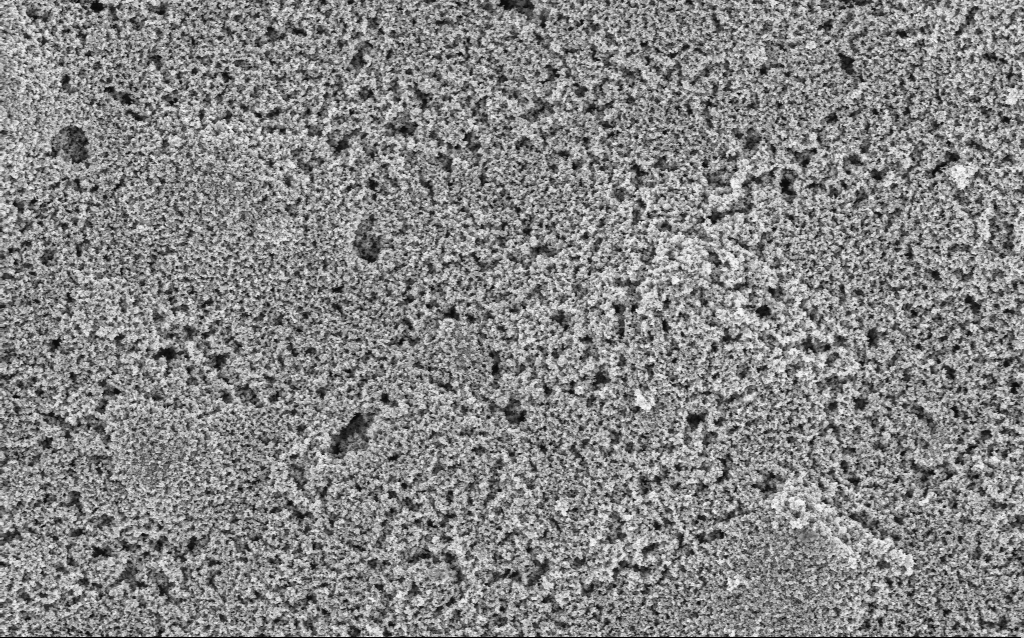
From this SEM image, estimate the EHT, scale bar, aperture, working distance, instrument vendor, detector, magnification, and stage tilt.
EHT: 3 kV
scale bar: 1000 nm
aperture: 30 µm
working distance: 7.6 mm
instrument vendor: Zeiss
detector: InLens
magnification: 23.9 K X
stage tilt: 0°